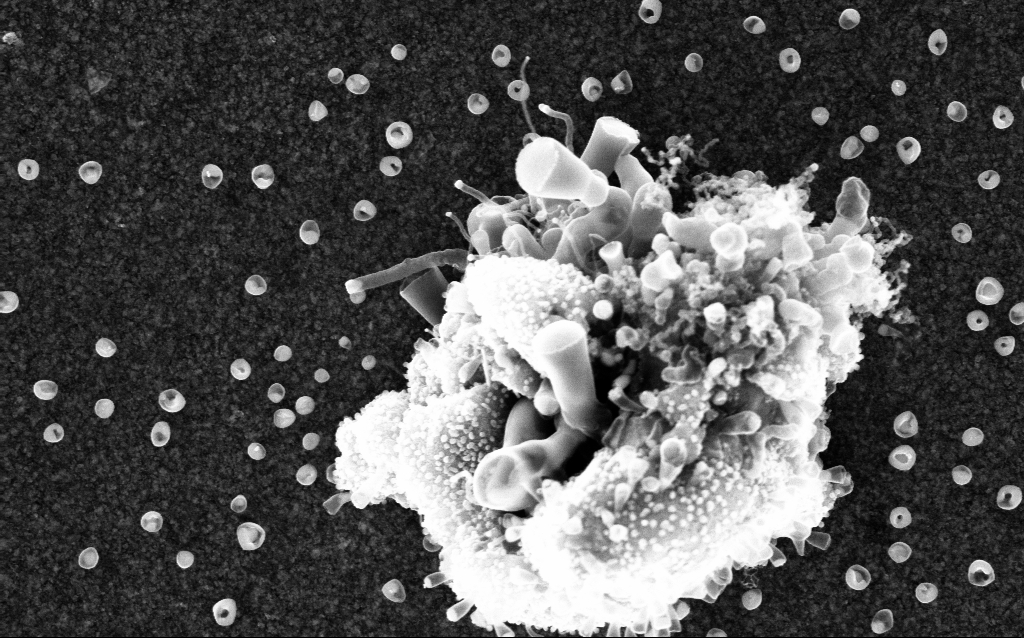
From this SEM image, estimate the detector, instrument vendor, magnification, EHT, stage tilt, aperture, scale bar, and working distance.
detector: InLens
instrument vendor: Zeiss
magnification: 75.68 K X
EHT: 5 kV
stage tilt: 0°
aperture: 30 µm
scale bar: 200 nm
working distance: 2.8 mm